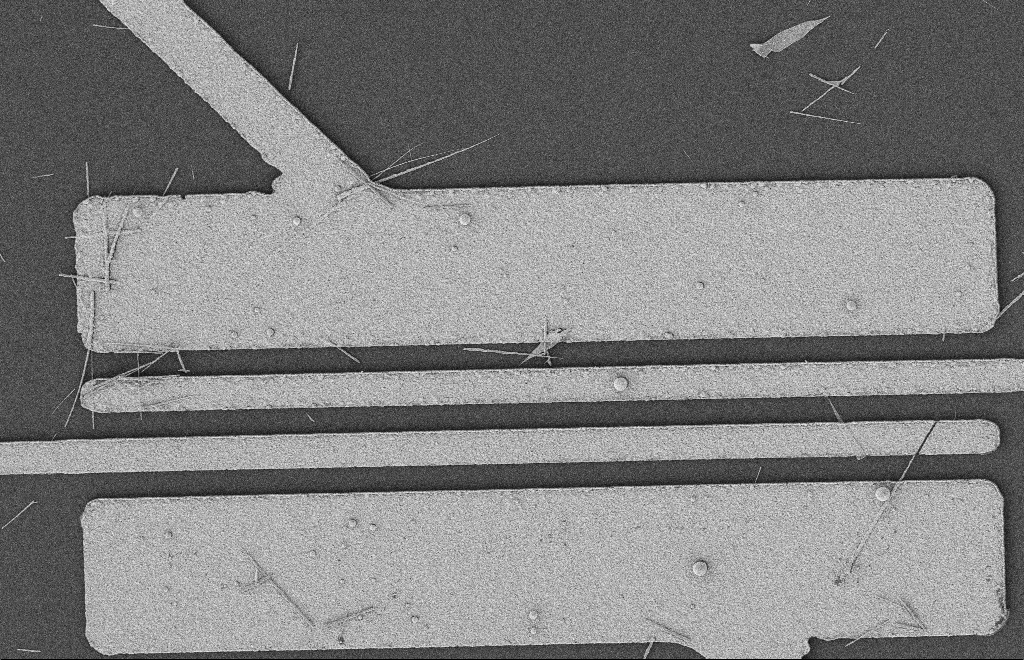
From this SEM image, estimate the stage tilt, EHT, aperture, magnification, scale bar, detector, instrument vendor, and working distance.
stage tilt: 0°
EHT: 2 kV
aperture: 20 µm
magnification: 5.5 K X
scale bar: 2000 nm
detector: SE2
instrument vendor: Zeiss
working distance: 12 mm